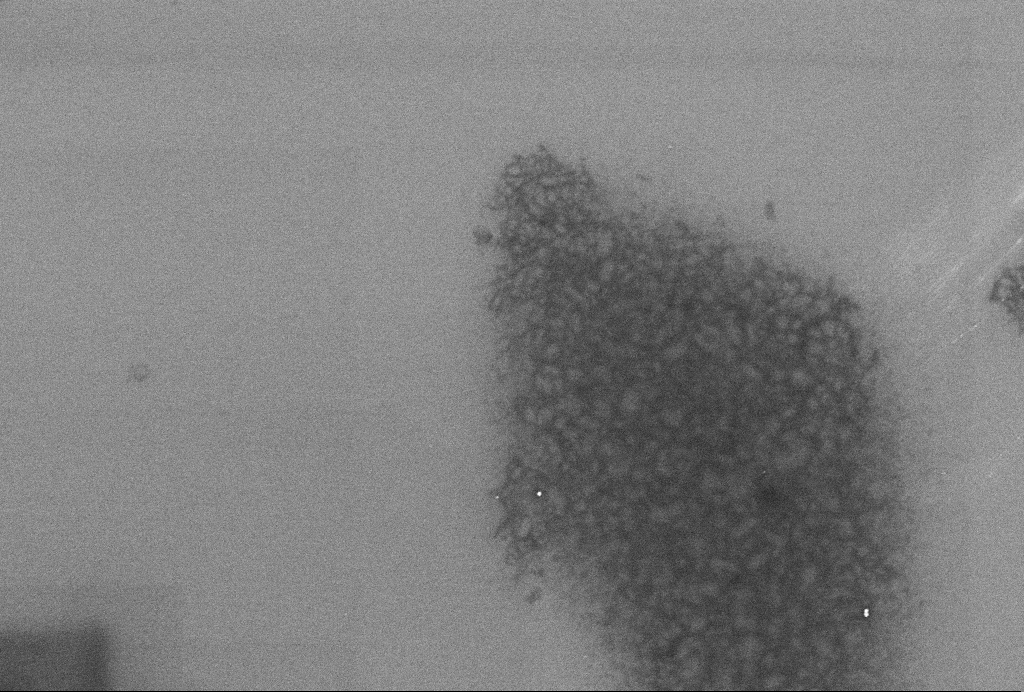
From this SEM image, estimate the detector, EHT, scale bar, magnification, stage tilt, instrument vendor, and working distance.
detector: InLens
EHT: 2 kV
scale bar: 1000 nm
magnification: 56.73 K X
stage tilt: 0°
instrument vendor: Zeiss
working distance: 3.3 mm